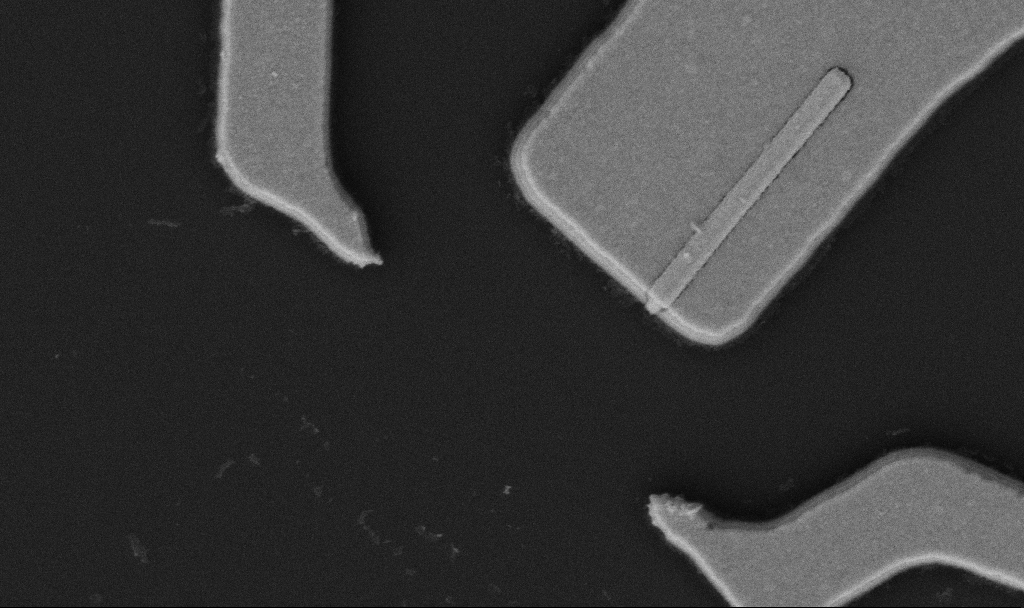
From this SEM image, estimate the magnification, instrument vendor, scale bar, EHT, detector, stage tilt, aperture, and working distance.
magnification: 50 K X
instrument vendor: Zeiss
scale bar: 1000 nm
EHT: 5 kV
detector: SE2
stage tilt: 0°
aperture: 30 µm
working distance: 10.7 mm